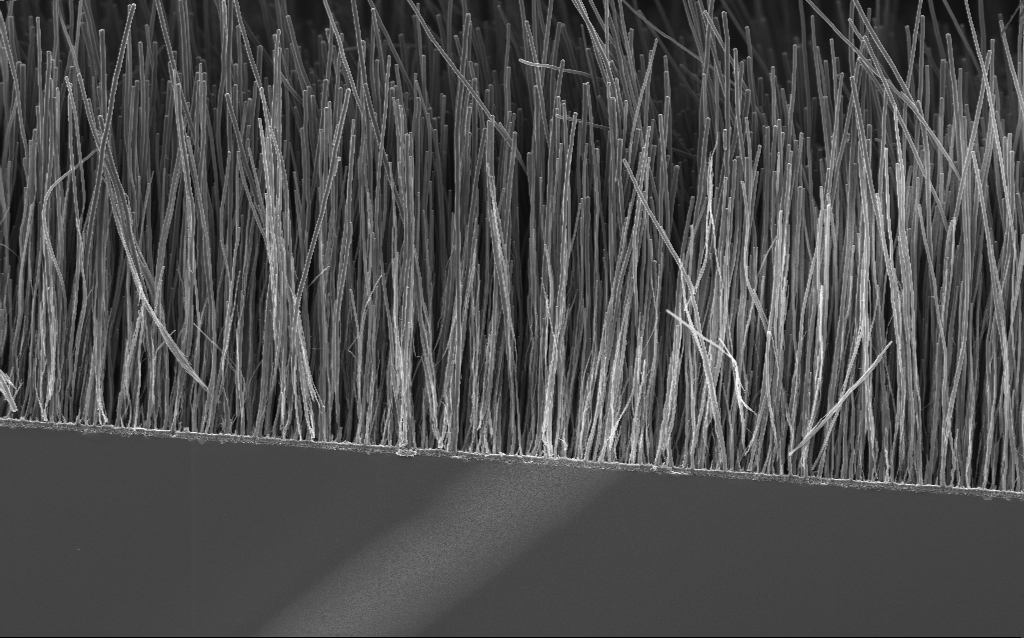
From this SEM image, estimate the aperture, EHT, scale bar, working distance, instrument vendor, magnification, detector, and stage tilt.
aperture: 30 µm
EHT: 3 kV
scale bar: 2000 nm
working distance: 3 mm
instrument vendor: Zeiss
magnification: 8.26 K X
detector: InLens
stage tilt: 0°